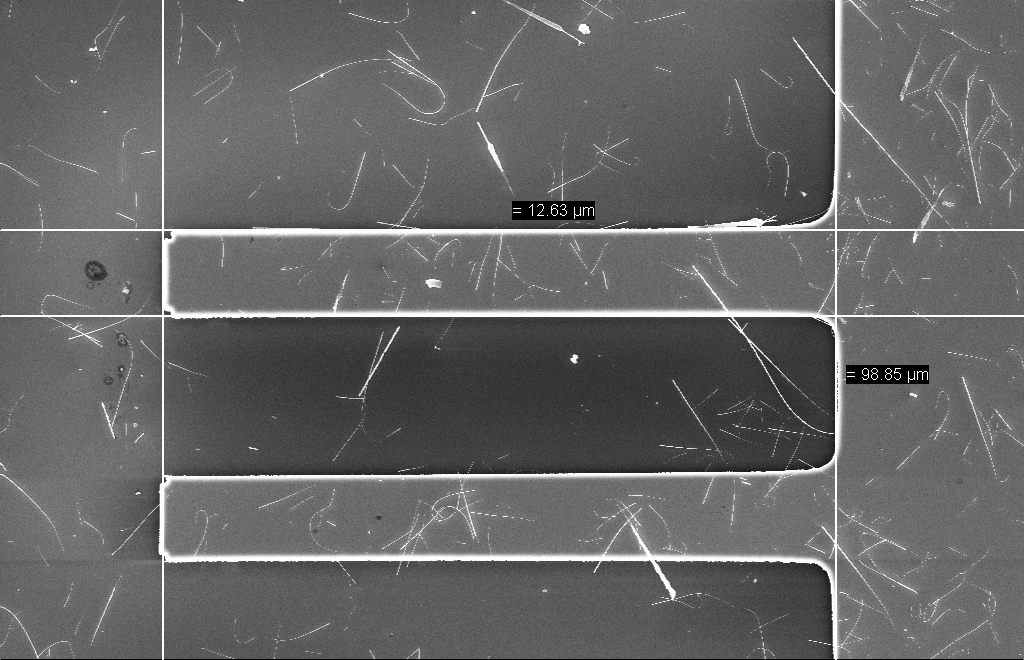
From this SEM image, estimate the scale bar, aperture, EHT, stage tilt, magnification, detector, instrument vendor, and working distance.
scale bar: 10000 nm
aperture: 20 µm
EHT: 10 kV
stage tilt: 0°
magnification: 2.5 K X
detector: InLens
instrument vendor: Zeiss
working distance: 6 mm